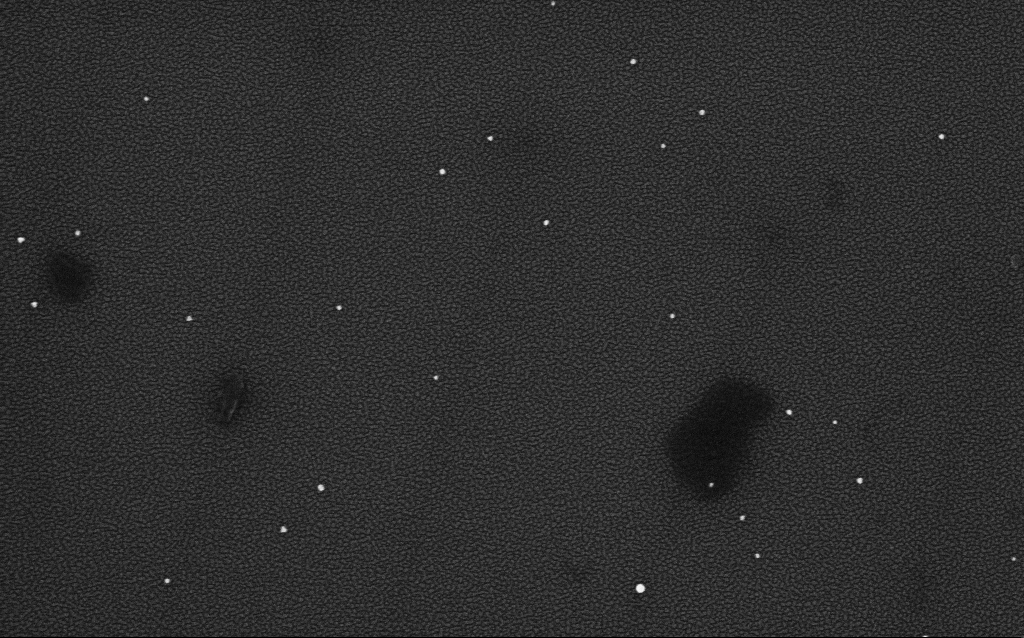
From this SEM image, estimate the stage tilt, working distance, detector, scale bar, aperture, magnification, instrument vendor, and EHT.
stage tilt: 0°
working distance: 2.1 mm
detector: InLens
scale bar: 200 nm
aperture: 30 µm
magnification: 100 K X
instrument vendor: Zeiss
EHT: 4 kV